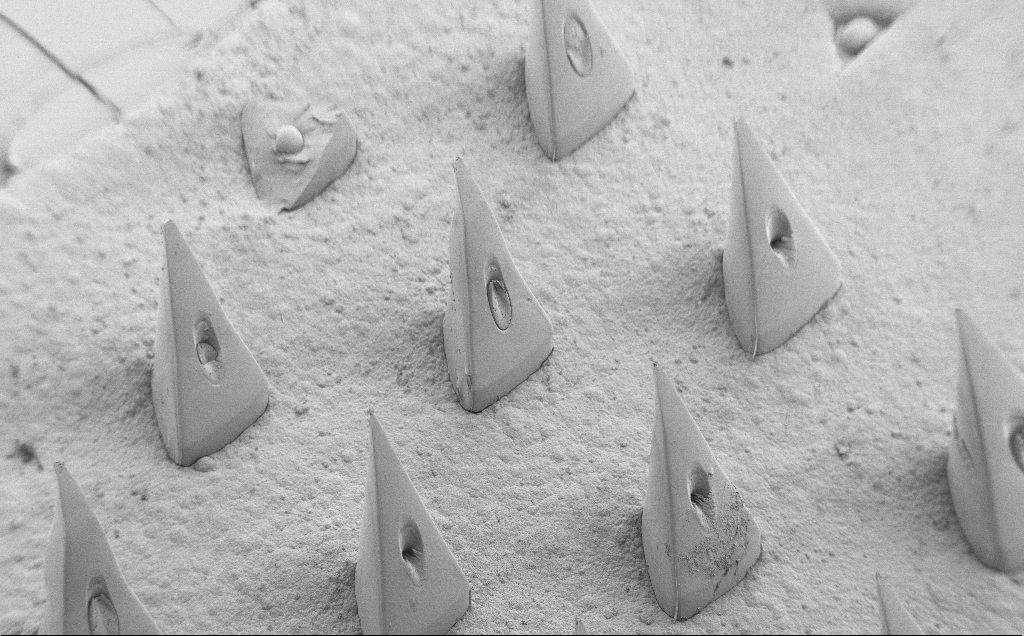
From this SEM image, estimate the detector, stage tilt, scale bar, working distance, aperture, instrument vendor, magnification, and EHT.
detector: SE2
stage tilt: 40°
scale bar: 200000 nm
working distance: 10 mm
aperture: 30 µm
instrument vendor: Zeiss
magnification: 0.073 K X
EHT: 5 kV